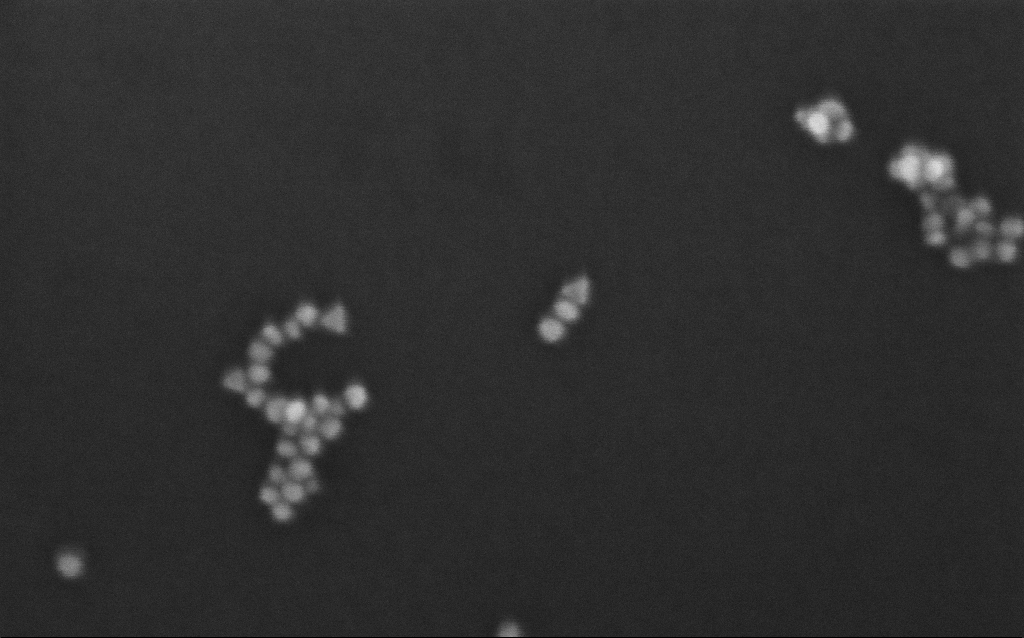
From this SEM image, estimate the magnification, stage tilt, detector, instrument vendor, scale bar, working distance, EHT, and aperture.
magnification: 500 K X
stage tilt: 0°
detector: InLens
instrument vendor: Zeiss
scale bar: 100 nm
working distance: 7 mm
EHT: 10 kV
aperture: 30 µm